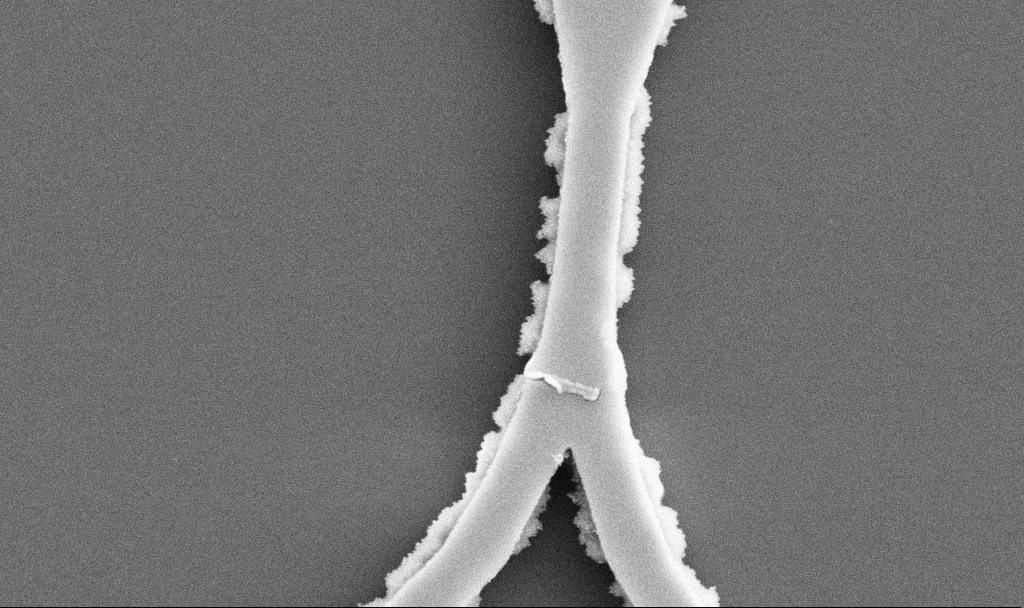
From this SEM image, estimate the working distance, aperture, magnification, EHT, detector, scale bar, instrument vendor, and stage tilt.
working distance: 7.4 mm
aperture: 30 µm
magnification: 44.13 K X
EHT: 5 kV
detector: SE2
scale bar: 1000 nm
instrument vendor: Zeiss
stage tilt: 0°